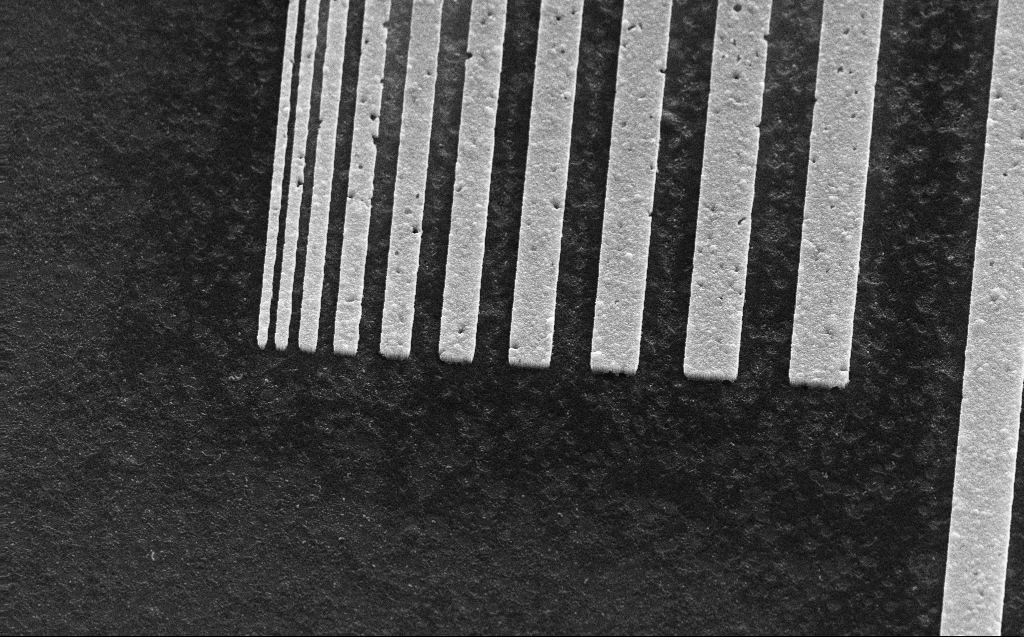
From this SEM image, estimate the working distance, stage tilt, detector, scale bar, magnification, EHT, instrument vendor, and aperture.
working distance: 5 mm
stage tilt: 45°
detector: SE2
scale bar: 1000 nm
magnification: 21.99 K X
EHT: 30 kV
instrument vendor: Zeiss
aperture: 30 µm